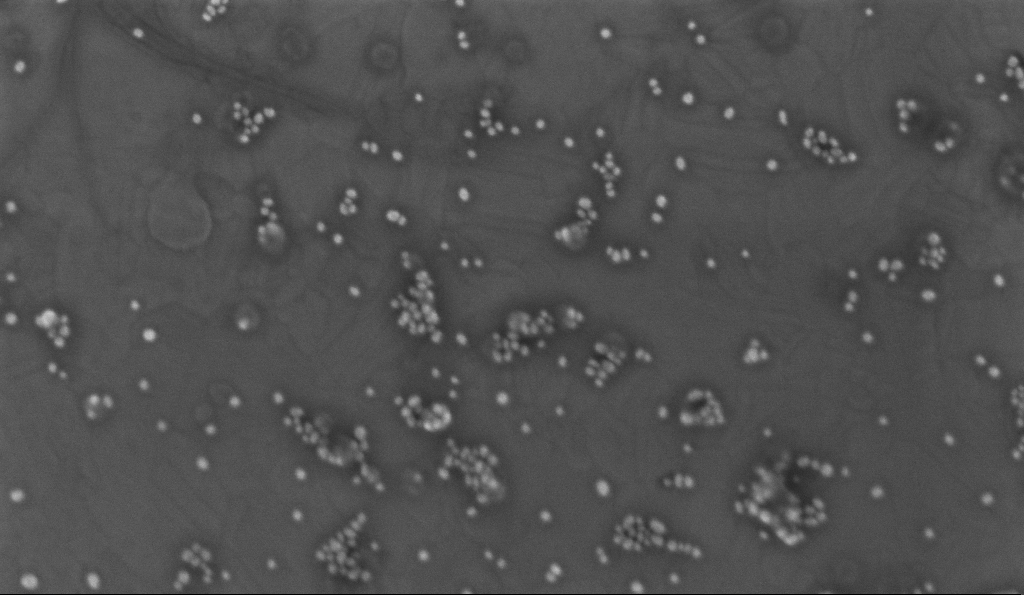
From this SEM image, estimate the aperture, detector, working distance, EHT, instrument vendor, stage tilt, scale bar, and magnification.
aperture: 30 µm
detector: InLens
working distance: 3.2 mm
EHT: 2 kV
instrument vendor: Zeiss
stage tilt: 0°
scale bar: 200 nm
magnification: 150 K X